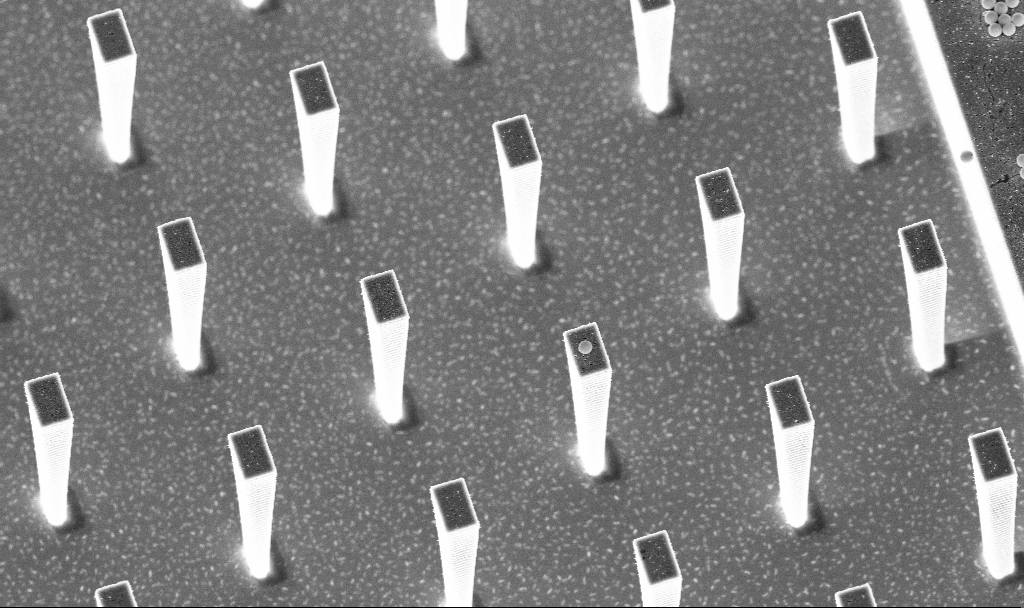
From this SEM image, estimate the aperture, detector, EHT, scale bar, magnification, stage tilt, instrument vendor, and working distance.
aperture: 30 µm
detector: InLens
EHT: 5 kV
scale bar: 10000 nm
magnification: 6.98 K X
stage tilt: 20°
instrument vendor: Zeiss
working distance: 5.2 mm